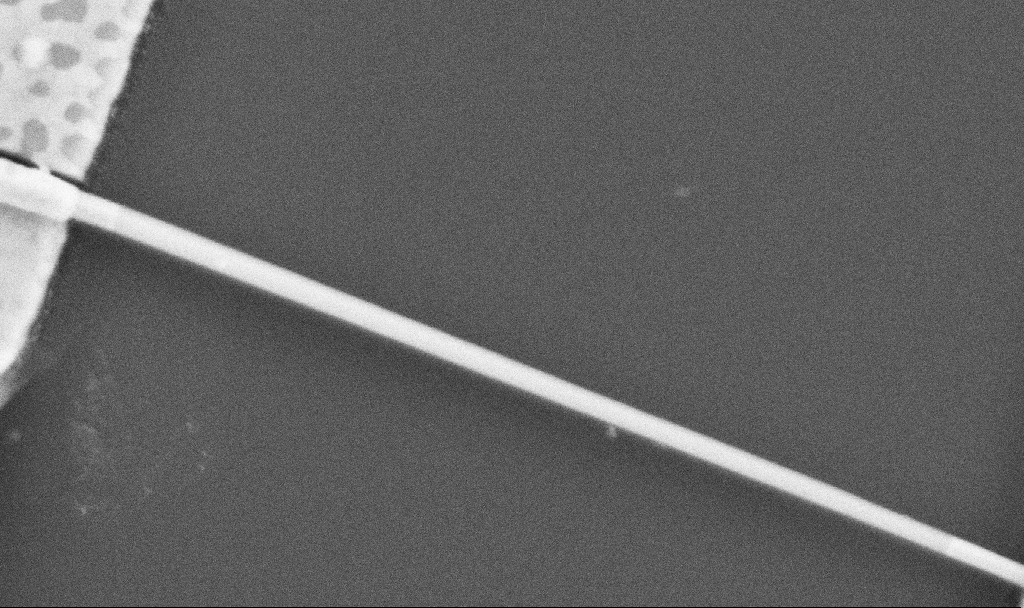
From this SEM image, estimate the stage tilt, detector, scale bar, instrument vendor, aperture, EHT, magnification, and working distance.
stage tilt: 0°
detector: SE2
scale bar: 200 nm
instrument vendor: Zeiss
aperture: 30 µm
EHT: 5 kV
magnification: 100 K X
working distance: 10.5 mm